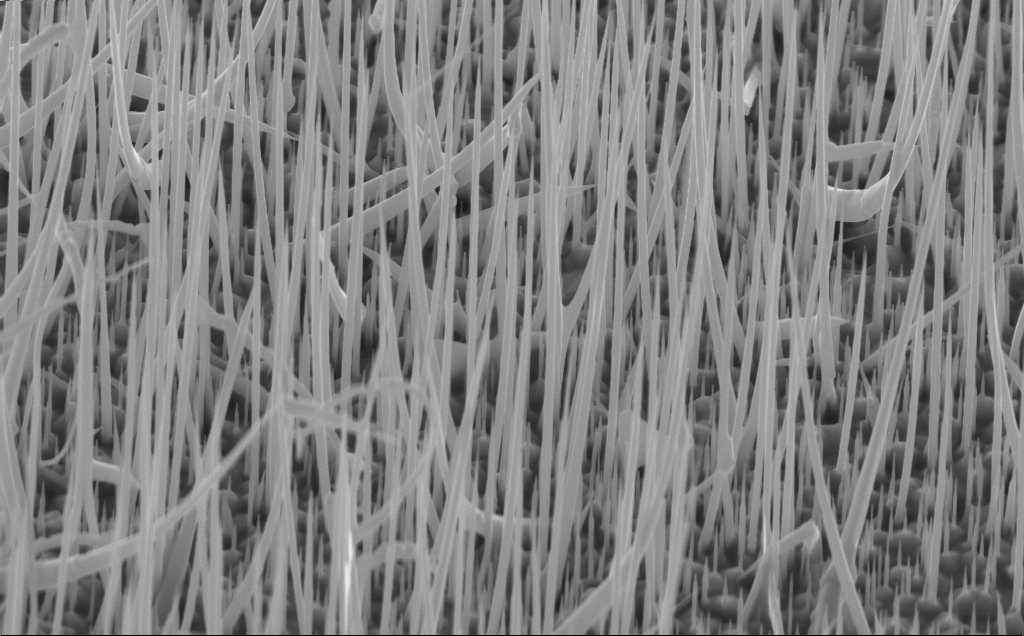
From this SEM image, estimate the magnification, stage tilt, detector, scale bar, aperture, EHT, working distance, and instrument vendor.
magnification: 25.76 K X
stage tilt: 45°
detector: InLens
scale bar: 1000 nm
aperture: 30 µm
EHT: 10 kV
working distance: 4 mm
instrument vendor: Zeiss